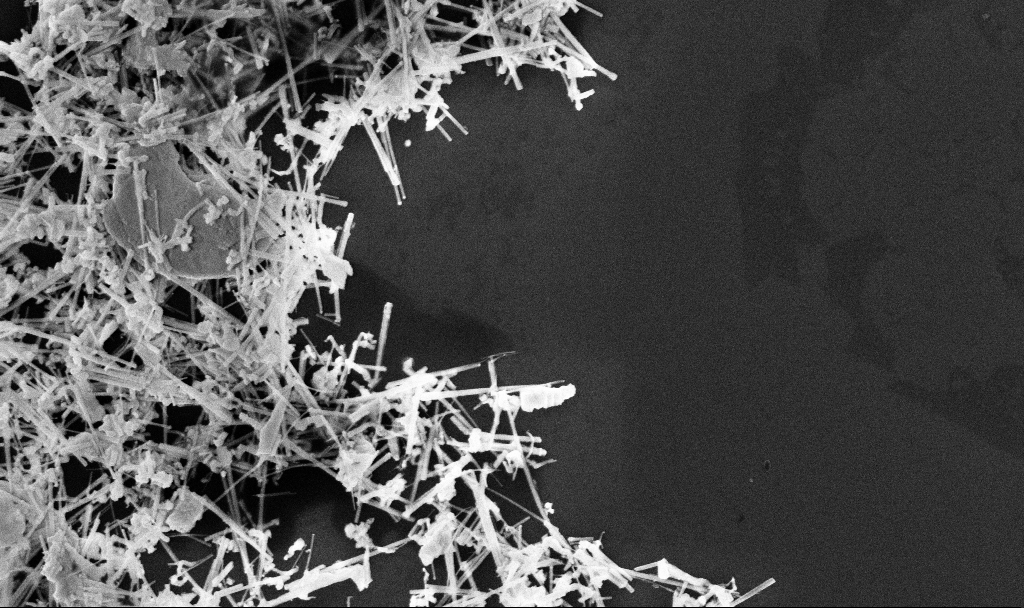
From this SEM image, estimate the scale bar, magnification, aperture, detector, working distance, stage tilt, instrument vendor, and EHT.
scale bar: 1000 nm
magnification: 37.27 K X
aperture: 30 µm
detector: InLens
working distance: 3.3 mm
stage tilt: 0°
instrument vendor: Zeiss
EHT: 5 kV